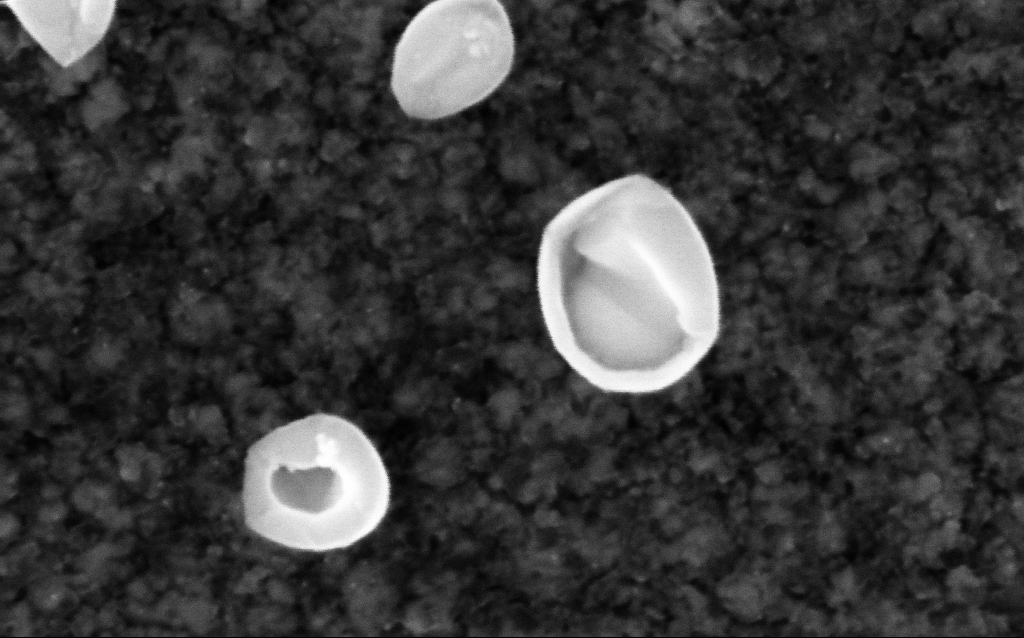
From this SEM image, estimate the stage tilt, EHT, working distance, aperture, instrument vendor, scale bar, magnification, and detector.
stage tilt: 0°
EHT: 5 kV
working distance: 2.8 mm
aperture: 30 µm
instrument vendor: Zeiss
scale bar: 100 nm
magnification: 436.32 K X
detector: InLens